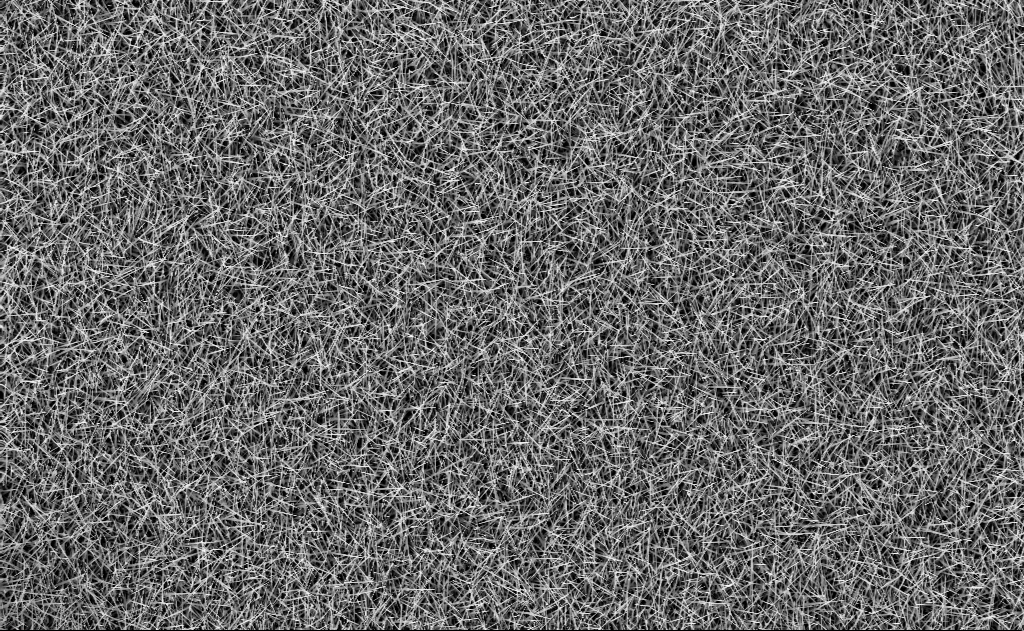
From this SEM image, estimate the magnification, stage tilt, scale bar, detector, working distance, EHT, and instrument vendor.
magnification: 5 K X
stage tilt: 0°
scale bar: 10000 nm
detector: InLens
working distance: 7 mm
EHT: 10 kV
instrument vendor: Zeiss